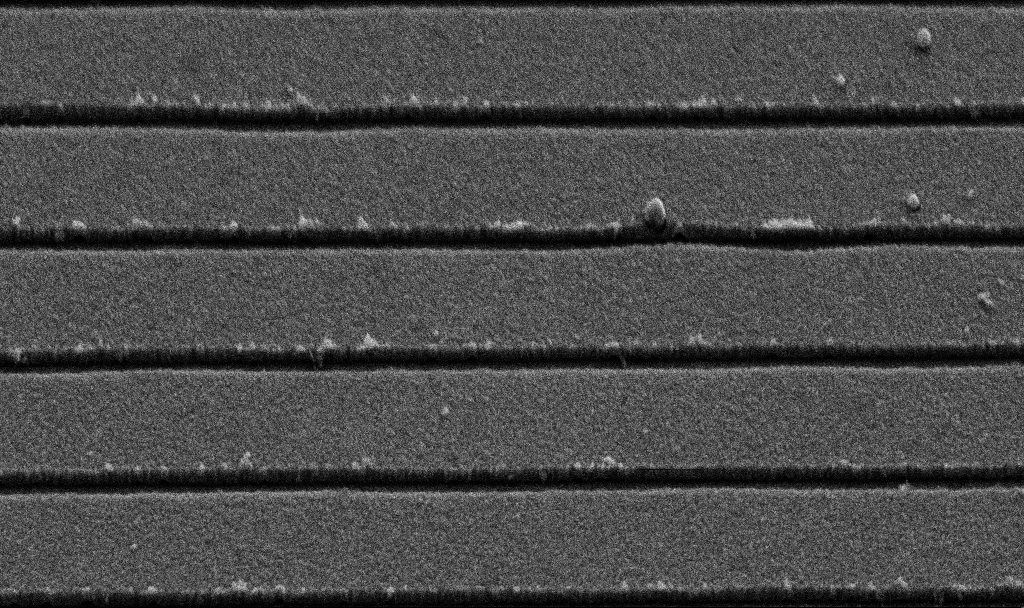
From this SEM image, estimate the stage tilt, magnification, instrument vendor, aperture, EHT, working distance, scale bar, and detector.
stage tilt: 45°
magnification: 57.29 K X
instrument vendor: Zeiss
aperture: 30 µm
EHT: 5 kV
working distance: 8.7 mm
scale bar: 1000 nm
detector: SE2